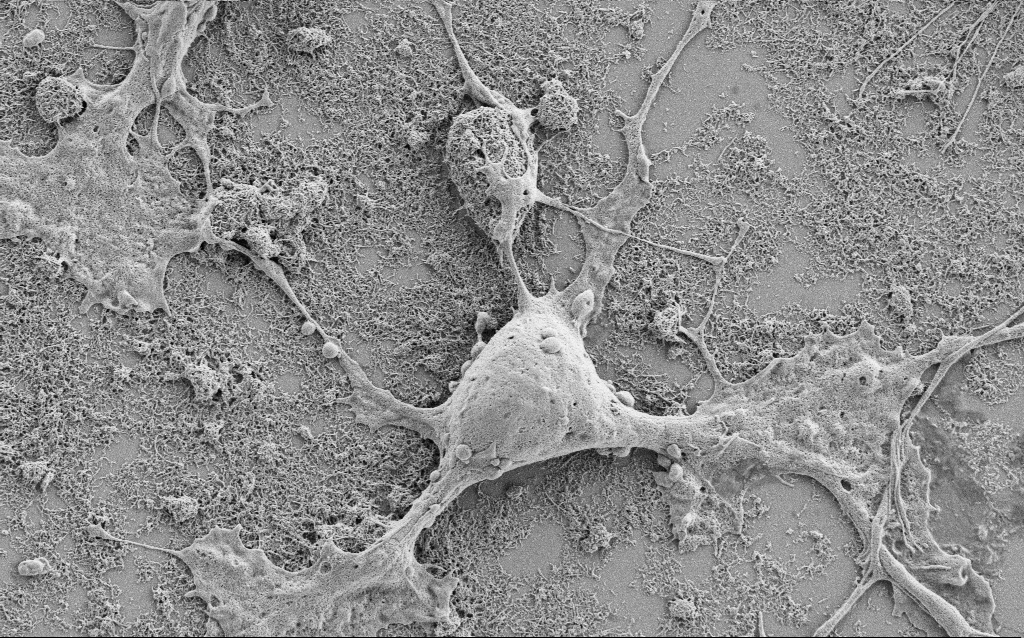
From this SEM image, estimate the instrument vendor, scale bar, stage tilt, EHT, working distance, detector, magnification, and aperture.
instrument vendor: Zeiss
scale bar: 2000 nm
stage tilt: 0°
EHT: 1.5 kV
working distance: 6.9 mm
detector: SE2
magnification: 7.5 K X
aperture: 30 µm